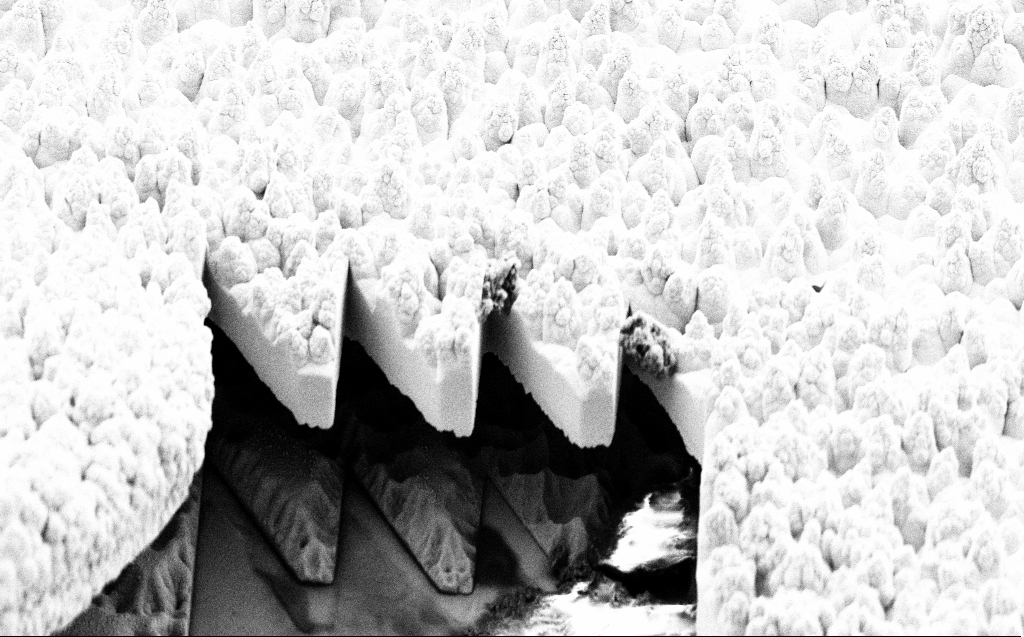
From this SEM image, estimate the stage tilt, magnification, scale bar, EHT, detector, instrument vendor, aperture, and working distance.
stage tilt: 45.1°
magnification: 4.54 K X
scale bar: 10000 nm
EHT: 5 kV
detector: SE2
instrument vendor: Zeiss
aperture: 30 µm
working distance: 9 mm